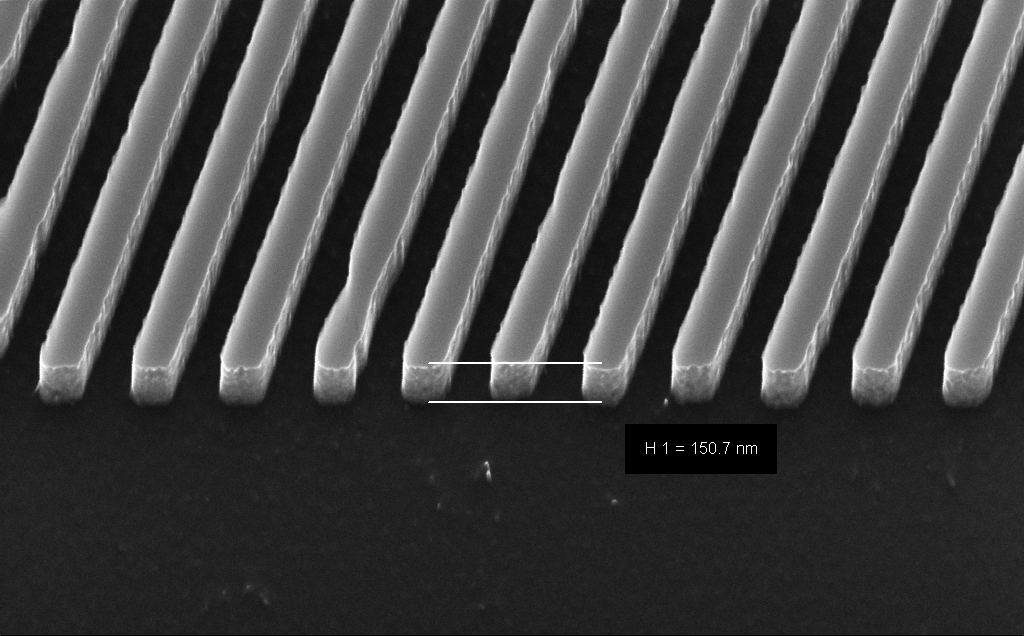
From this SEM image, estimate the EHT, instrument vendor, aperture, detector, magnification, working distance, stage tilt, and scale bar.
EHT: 10 kV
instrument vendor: Zeiss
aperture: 30 µm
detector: InLens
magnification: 95.06 K X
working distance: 5 mm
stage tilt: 30°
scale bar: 200 nm